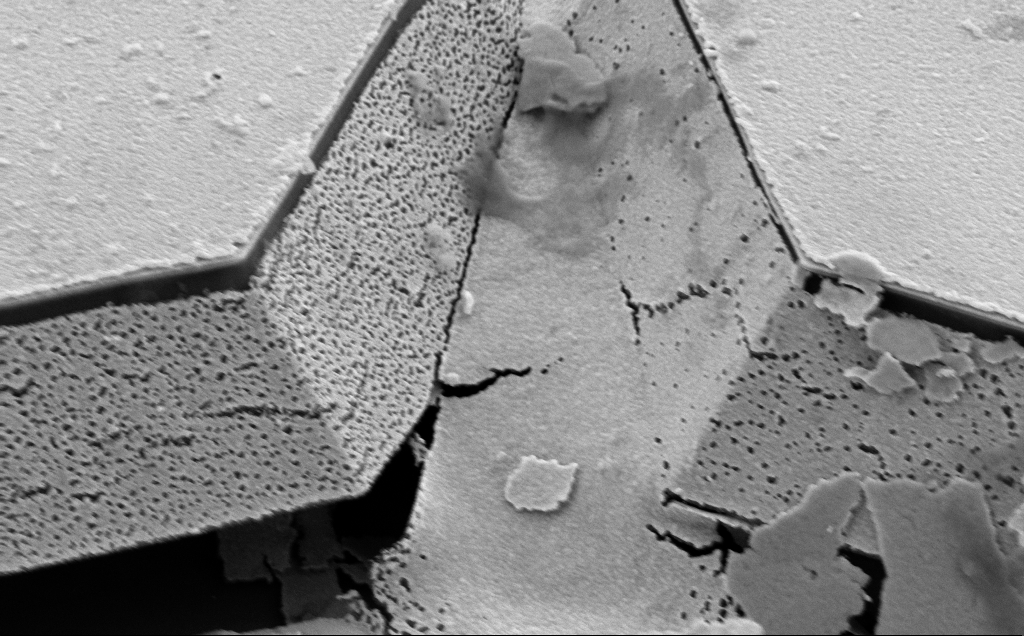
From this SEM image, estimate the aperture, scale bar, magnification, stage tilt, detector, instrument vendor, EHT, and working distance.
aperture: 30 µm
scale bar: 2000 nm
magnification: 32.32 K X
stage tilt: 50°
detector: SE2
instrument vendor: Zeiss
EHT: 5 kV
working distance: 10 mm